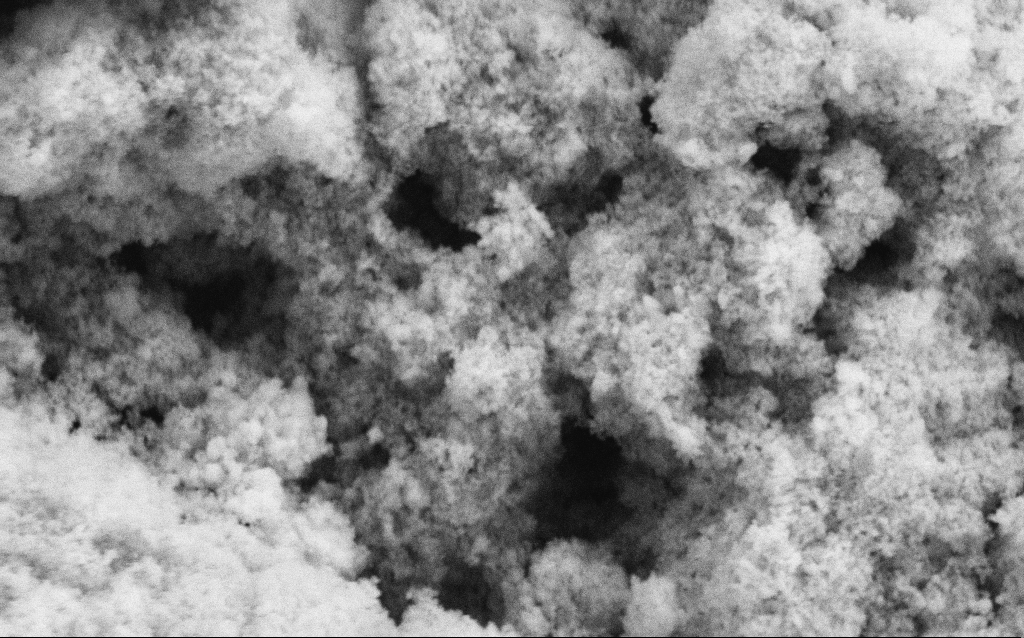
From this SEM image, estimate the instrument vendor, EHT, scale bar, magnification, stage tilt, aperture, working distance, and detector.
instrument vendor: Zeiss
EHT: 5 kV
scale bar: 1000 nm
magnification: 68.64 K X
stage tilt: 0°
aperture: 30 µm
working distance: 4.7 mm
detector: SE2